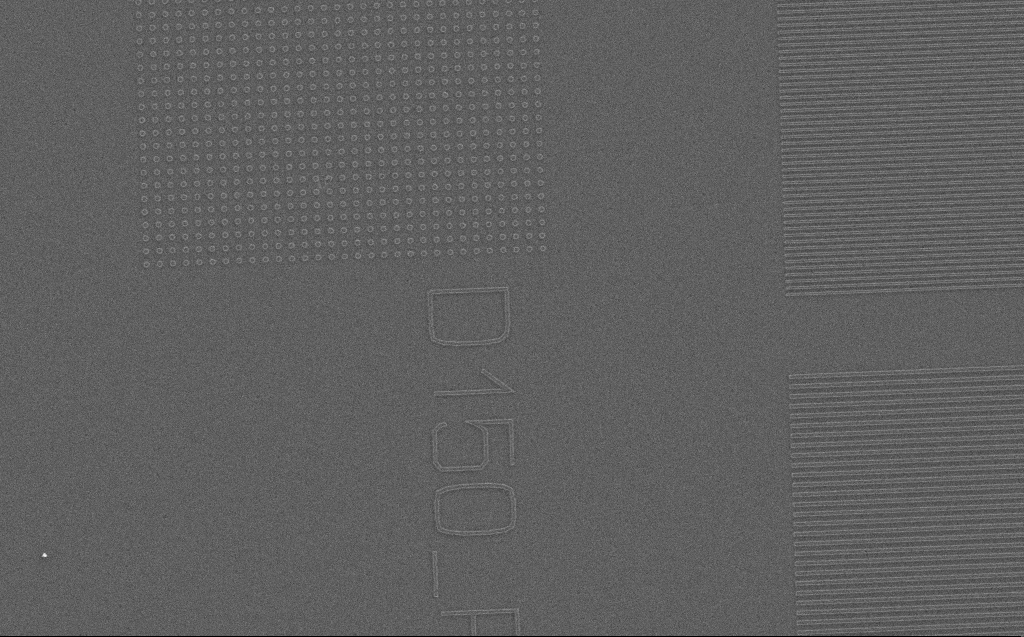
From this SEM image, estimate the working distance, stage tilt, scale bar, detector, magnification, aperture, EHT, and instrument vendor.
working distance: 4 mm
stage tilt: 0°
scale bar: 10000 nm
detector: SE2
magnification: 6.14 K X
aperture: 30 µm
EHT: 5 kV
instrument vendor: Zeiss